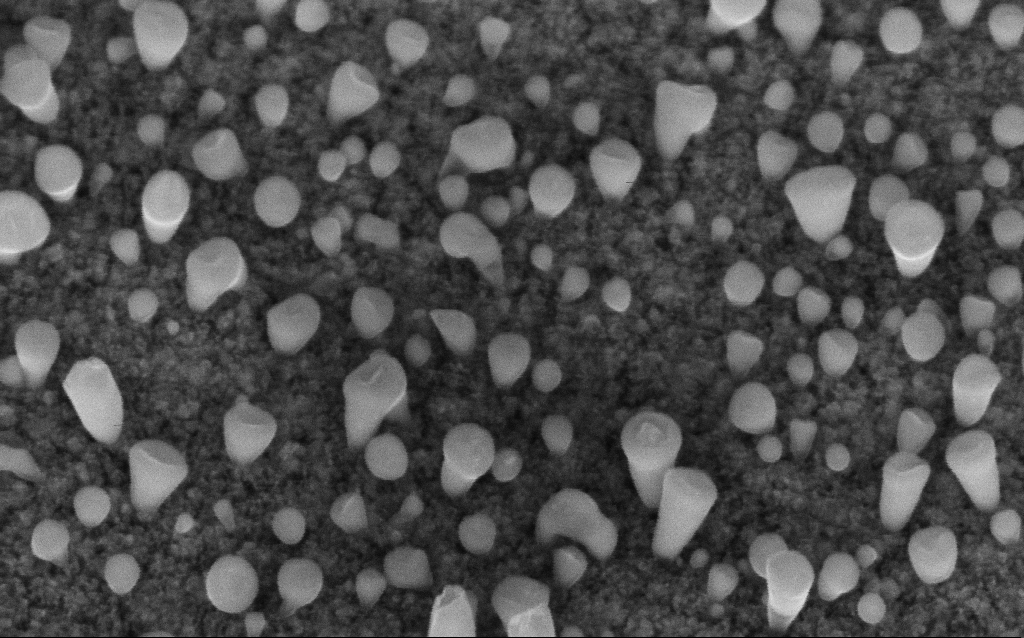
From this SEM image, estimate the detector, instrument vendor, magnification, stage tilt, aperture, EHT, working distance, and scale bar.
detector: InLens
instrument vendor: Zeiss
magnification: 200 K X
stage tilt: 45°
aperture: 30 µm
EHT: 5 kV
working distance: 6.2 mm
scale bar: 100 nm